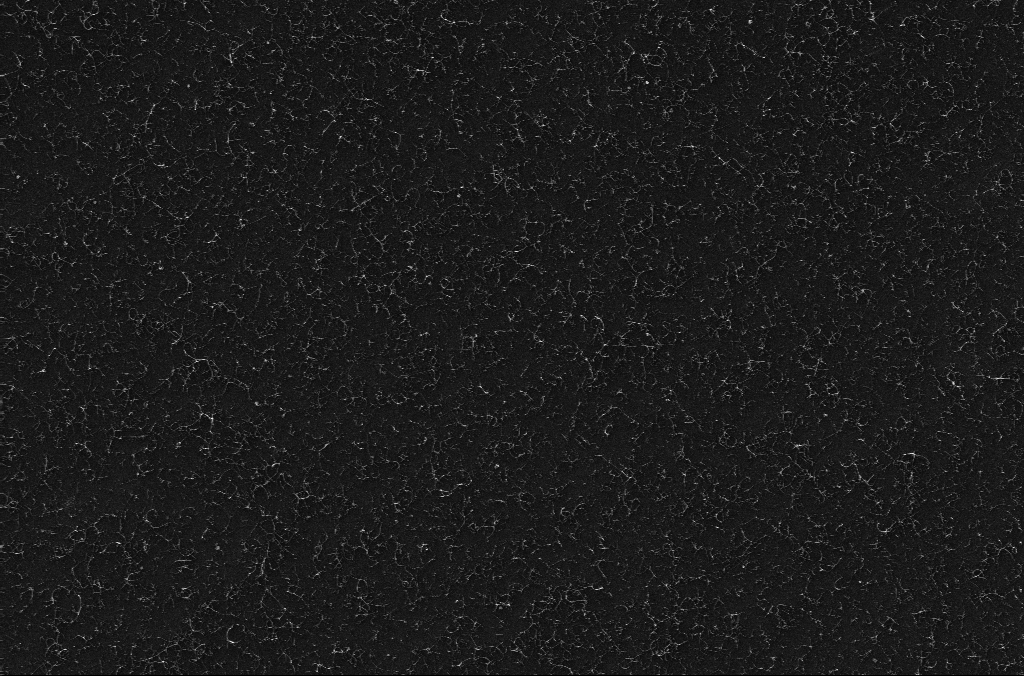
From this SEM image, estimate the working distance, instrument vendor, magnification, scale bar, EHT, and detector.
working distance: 3.2 mm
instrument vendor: Zeiss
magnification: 19.35 K X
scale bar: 2000 nm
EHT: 10 kV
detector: InLens